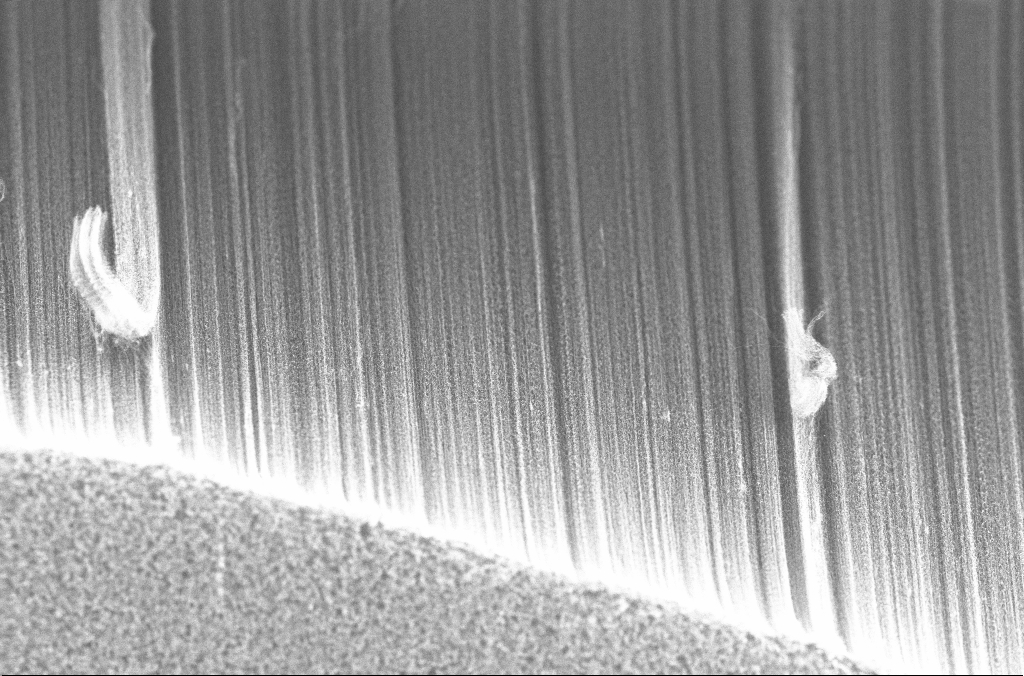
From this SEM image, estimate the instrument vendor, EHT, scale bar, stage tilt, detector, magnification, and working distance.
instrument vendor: Zeiss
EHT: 20 kV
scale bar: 10000 nm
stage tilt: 0°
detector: InLens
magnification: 2 K X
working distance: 4 mm